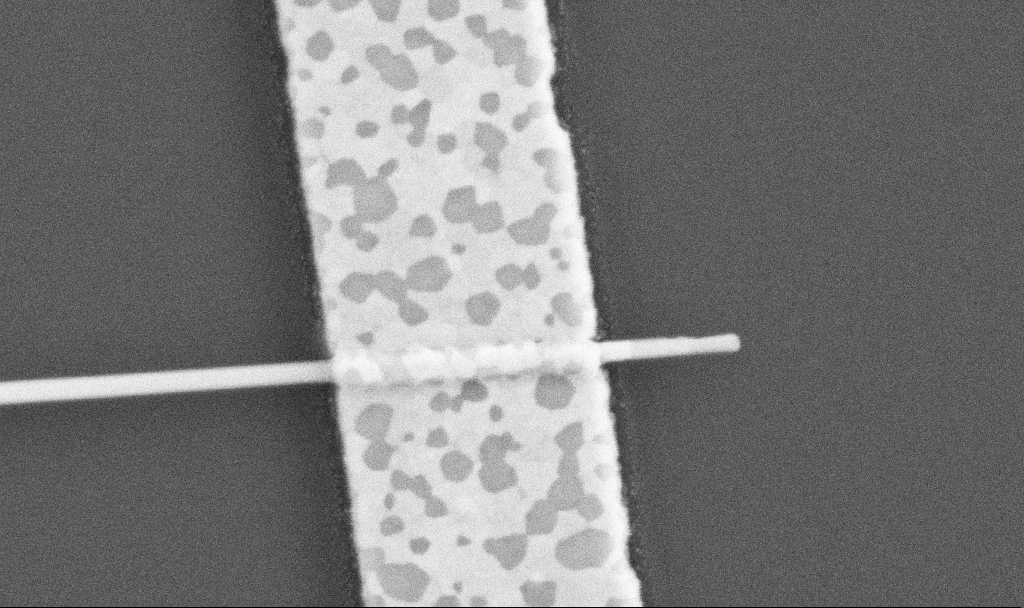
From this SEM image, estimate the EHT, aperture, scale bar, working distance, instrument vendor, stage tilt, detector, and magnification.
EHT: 5 kV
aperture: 30 µm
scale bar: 200 nm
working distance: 10.5 mm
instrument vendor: Zeiss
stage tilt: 0°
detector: SE2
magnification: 100 K X